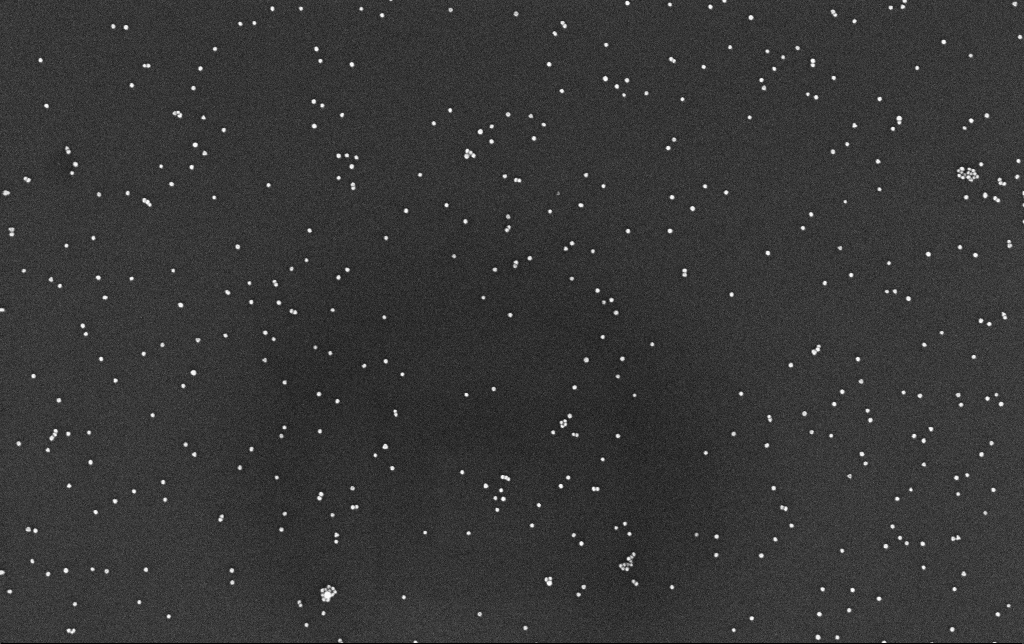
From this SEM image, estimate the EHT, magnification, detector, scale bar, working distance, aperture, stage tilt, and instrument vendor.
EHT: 10 kV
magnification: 100 K X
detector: InLens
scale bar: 200 nm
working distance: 3.4 mm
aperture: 30 µm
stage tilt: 0°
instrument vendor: Zeiss